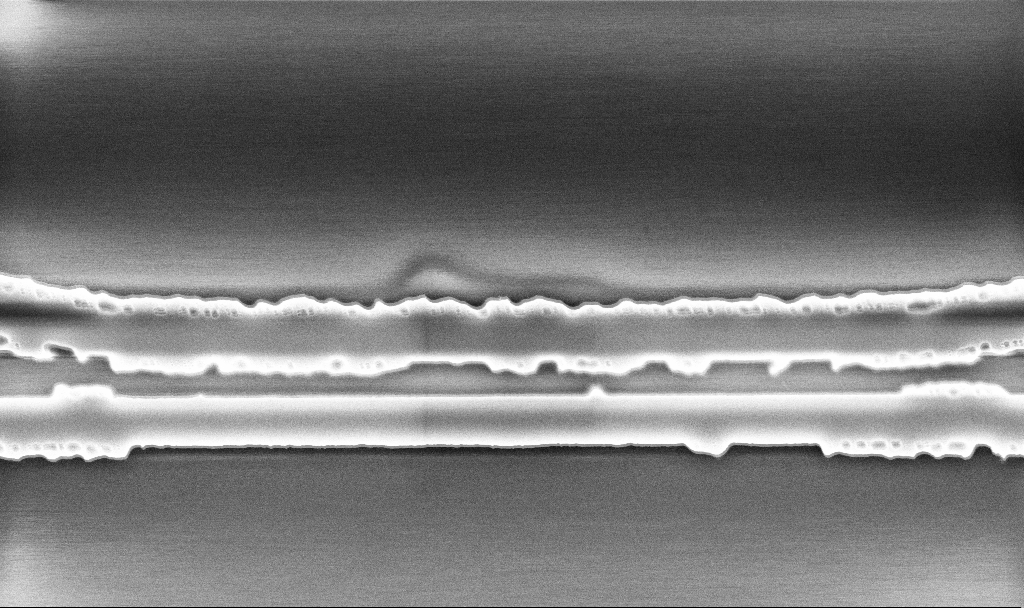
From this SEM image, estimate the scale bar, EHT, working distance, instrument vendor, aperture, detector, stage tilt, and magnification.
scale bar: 1000 nm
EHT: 5 kV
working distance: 10.1 mm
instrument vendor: Zeiss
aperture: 30 µm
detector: InLens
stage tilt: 0°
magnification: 37.38 K X